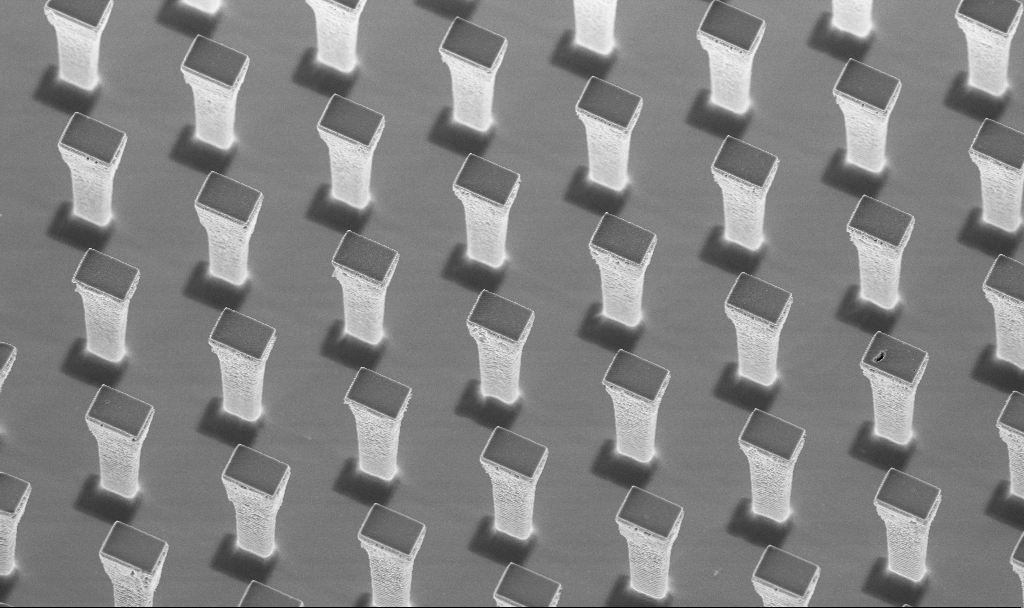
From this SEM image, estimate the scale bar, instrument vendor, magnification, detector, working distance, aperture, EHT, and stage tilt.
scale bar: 10000 nm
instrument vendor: Zeiss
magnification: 4.6 K X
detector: InLens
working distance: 4.2 mm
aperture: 30 µm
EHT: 5 kV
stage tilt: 20°